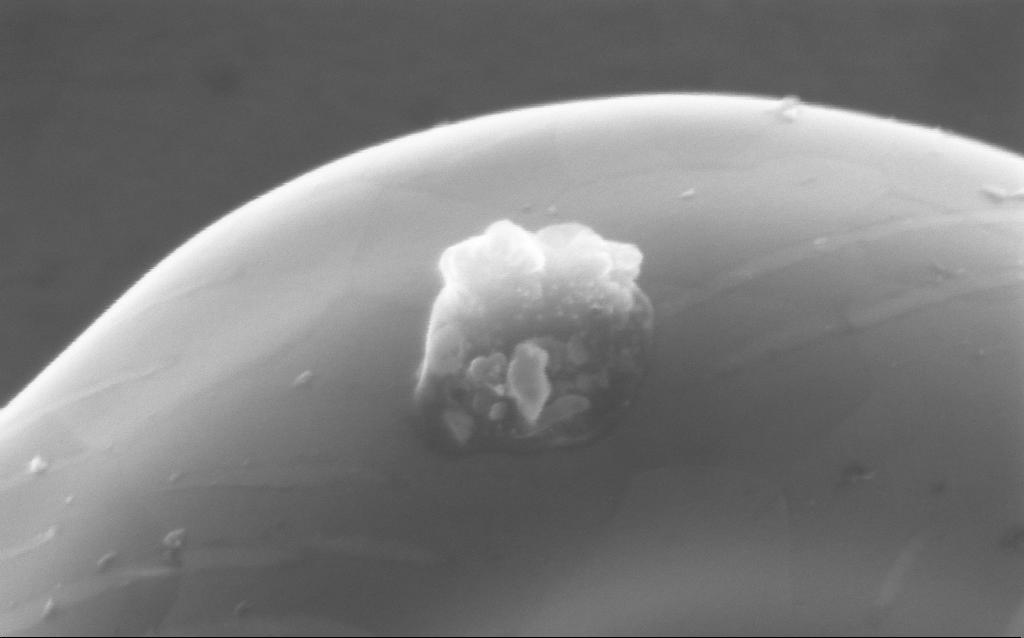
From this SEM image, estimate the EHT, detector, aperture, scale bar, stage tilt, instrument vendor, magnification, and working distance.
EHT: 5 kV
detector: InLens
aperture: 30 µm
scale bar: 200 nm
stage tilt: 0°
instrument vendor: Zeiss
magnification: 202.79 K X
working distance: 4 mm